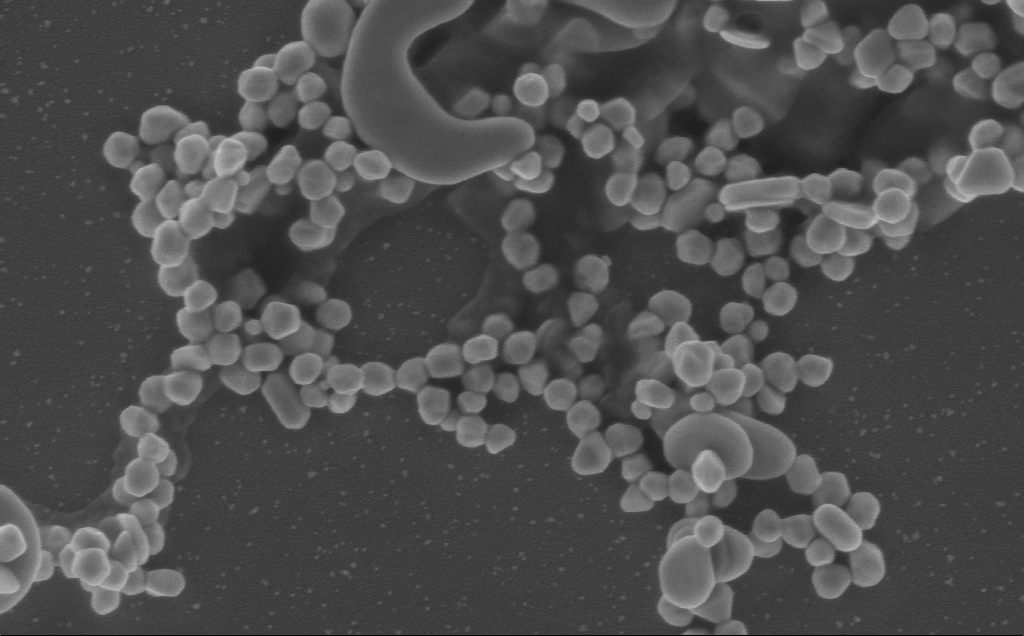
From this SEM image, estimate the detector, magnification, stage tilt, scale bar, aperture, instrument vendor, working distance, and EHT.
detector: InLens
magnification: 96.26 K X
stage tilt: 0°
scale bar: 200 nm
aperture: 30 µm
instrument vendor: Zeiss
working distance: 3 mm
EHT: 5 kV